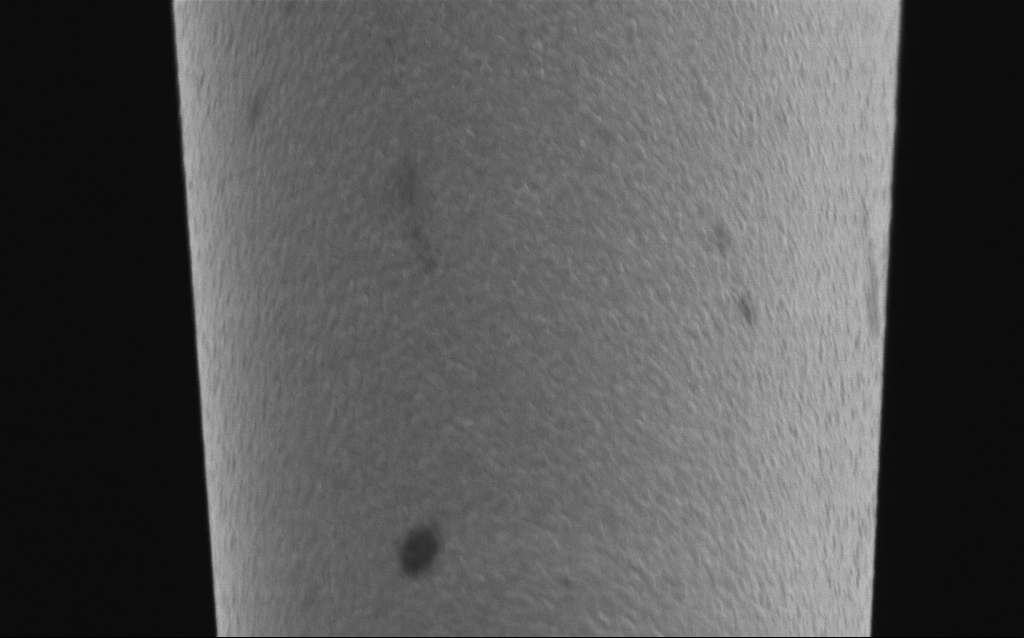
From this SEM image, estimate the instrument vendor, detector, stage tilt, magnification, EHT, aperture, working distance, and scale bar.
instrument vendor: Zeiss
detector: InLens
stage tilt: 44.9°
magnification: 50 K X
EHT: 2 kV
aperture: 30 µm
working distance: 6.3 mm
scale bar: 1000 nm